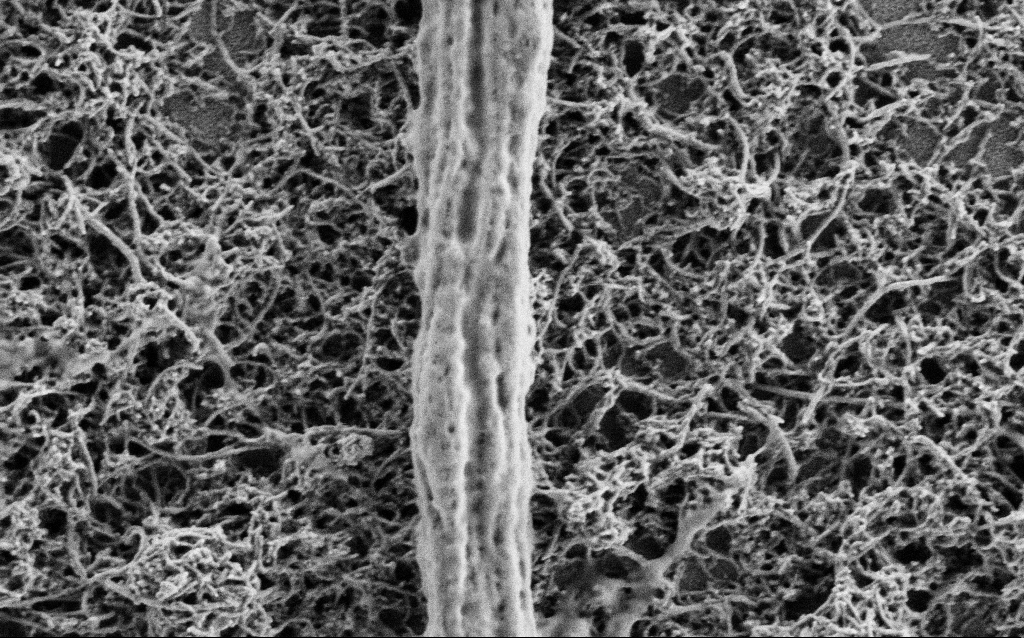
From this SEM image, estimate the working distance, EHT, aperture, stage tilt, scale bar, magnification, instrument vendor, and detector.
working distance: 4 mm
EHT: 1 kV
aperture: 30 µm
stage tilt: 0°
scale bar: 1000 nm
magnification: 50 K X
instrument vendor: Zeiss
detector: SE2